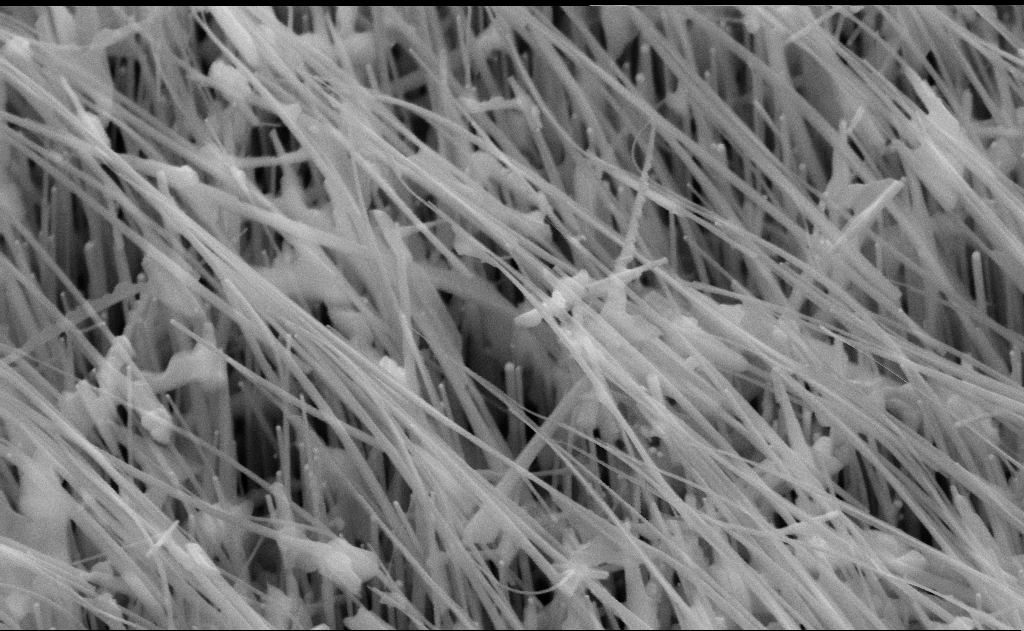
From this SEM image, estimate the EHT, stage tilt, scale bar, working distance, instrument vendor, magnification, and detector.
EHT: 10 kV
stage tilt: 45°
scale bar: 200 nm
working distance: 12 mm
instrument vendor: Zeiss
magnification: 80 K X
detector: SE2